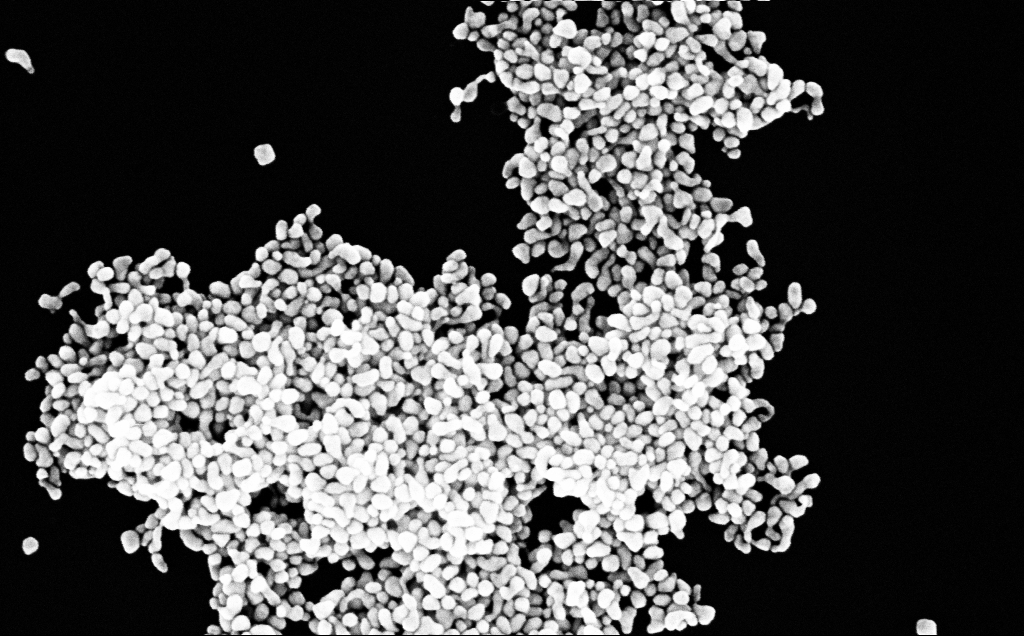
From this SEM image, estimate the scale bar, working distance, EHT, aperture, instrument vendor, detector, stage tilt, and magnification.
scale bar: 200 nm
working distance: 3.1 mm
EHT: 10 kV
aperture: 30 µm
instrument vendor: Zeiss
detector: InLens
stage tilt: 0°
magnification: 151.57 K X